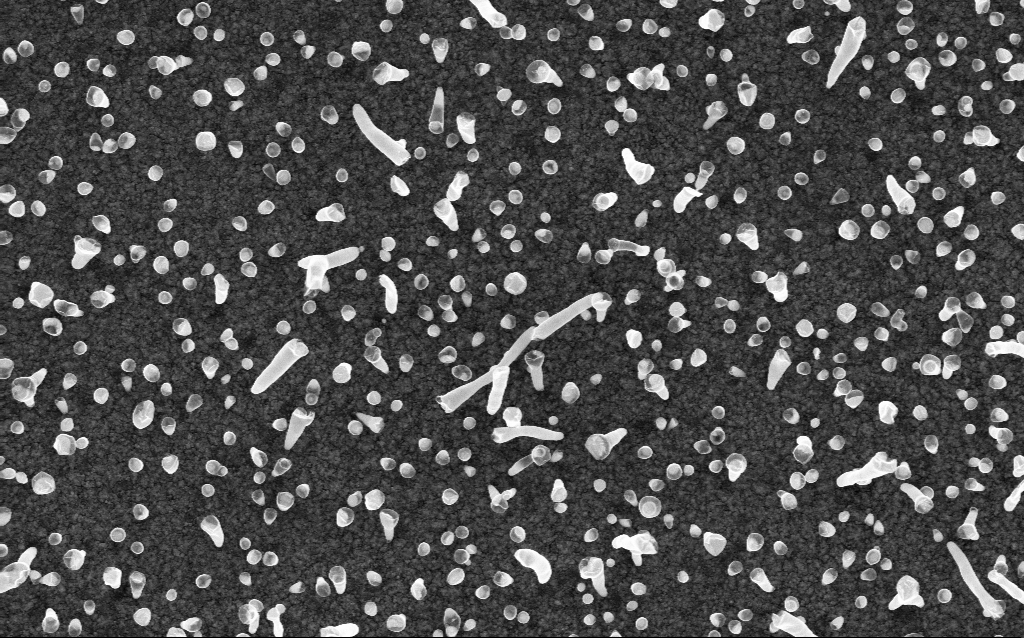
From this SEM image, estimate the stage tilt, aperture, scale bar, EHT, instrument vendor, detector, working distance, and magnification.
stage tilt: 0°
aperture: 30 µm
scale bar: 1000 nm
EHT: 5 kV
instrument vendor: Zeiss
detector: InLens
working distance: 2 mm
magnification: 50 K X